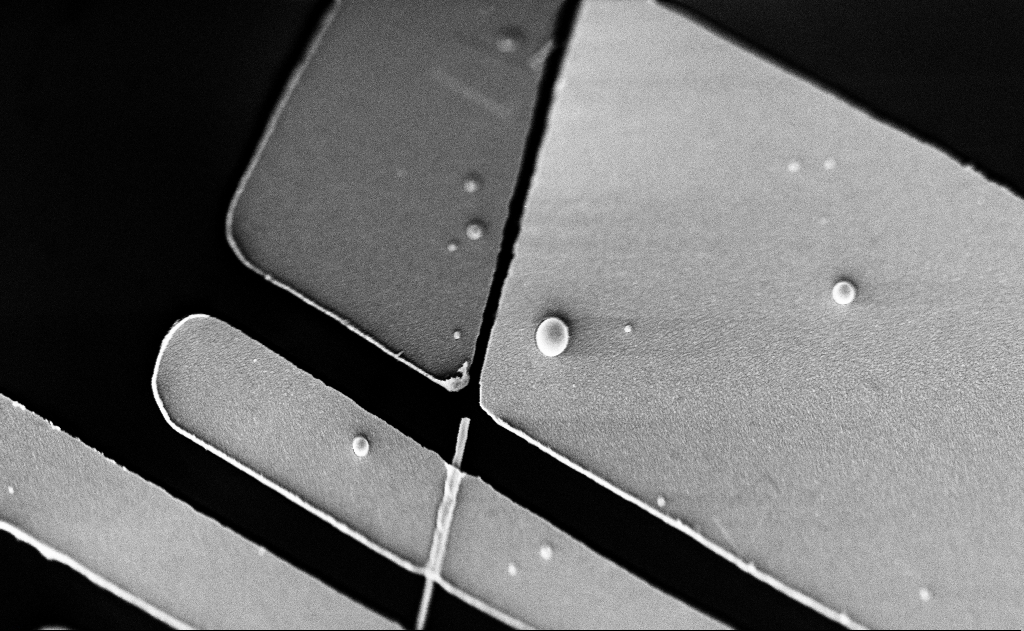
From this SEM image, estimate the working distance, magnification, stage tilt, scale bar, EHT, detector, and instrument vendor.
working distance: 20 mm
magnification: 14 K X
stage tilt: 0°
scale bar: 1000 nm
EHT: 5 kV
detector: SE2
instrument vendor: Zeiss